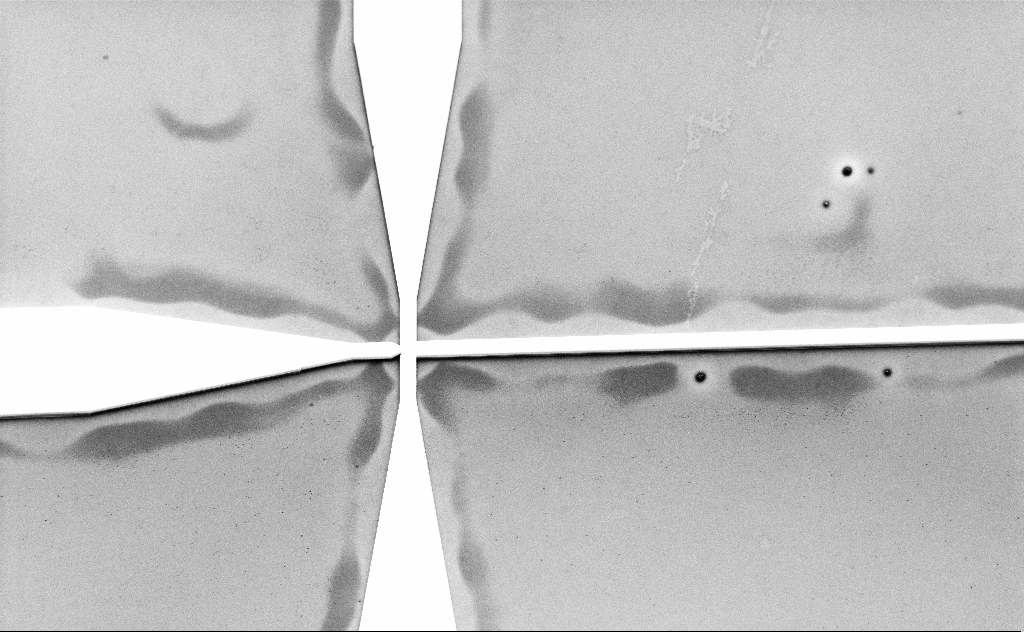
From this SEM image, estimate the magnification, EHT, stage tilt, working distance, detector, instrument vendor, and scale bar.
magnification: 0.474 K X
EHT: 3 kV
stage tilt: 45°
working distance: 12 mm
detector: SE2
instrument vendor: Zeiss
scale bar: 100000 nm